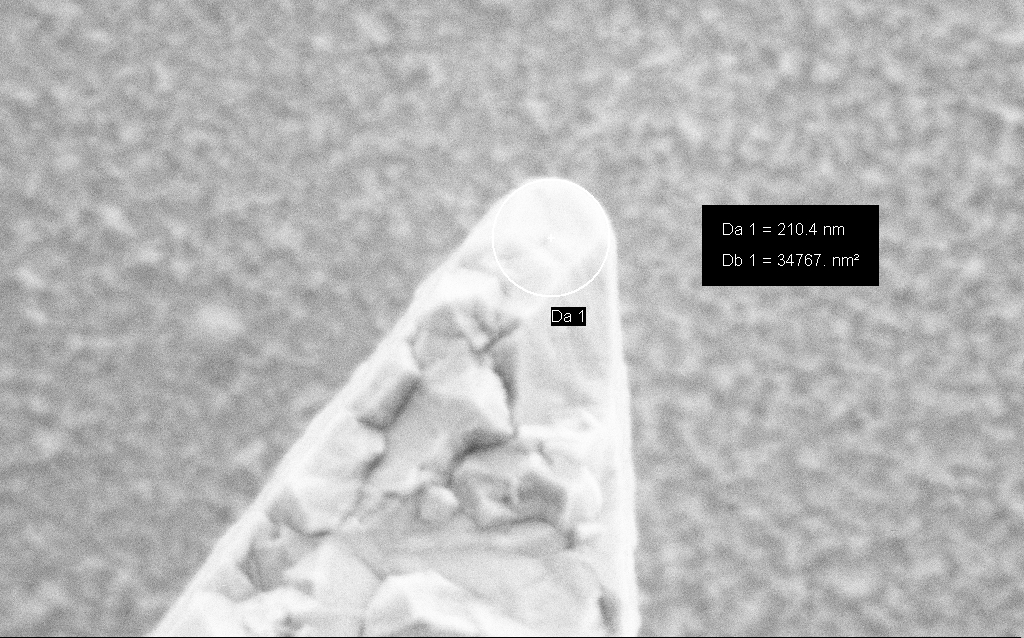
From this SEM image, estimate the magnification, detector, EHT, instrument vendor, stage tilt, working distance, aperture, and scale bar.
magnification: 205.94 K X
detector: SE2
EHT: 3 kV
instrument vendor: Zeiss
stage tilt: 0°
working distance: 4.5 mm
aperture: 30 µm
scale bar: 200 nm